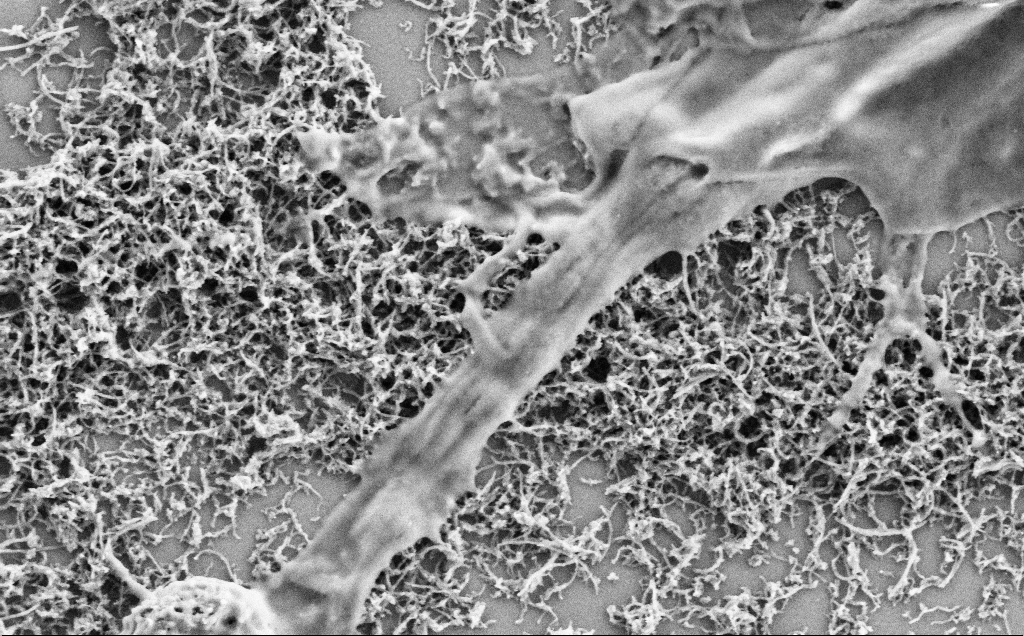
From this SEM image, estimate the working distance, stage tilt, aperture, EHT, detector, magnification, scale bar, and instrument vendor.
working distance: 7.1 mm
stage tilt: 0°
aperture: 30 µm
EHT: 2 kV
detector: SE2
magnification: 35 K X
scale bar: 2000 nm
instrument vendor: Zeiss